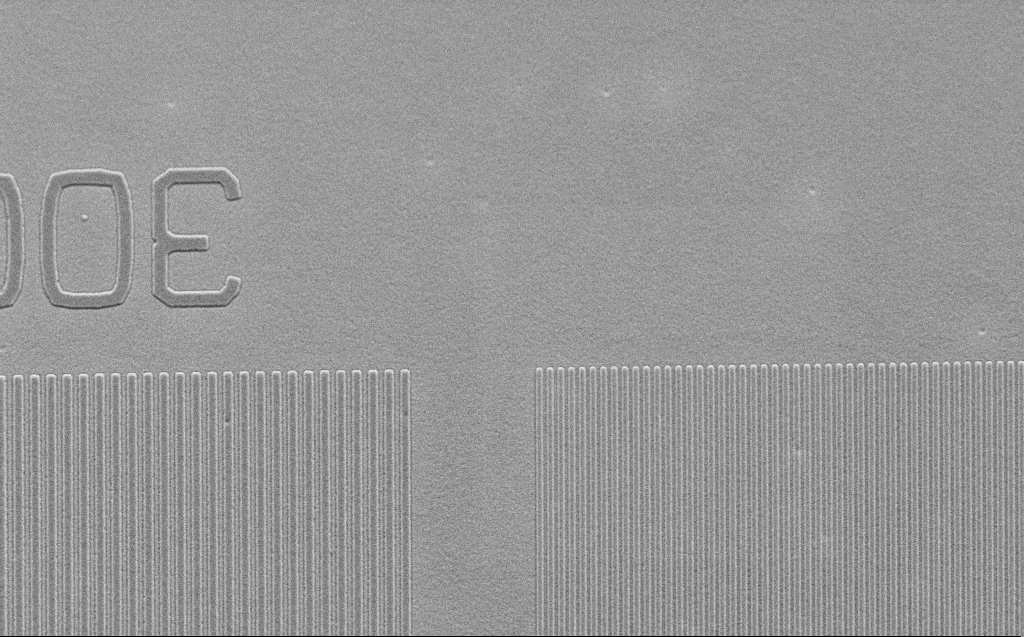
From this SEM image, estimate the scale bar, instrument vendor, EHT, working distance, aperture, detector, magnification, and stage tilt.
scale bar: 2000 nm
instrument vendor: Zeiss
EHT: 2.5 kV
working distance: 5 mm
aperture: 30 µm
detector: SE2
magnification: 9.92 K X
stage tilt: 30°